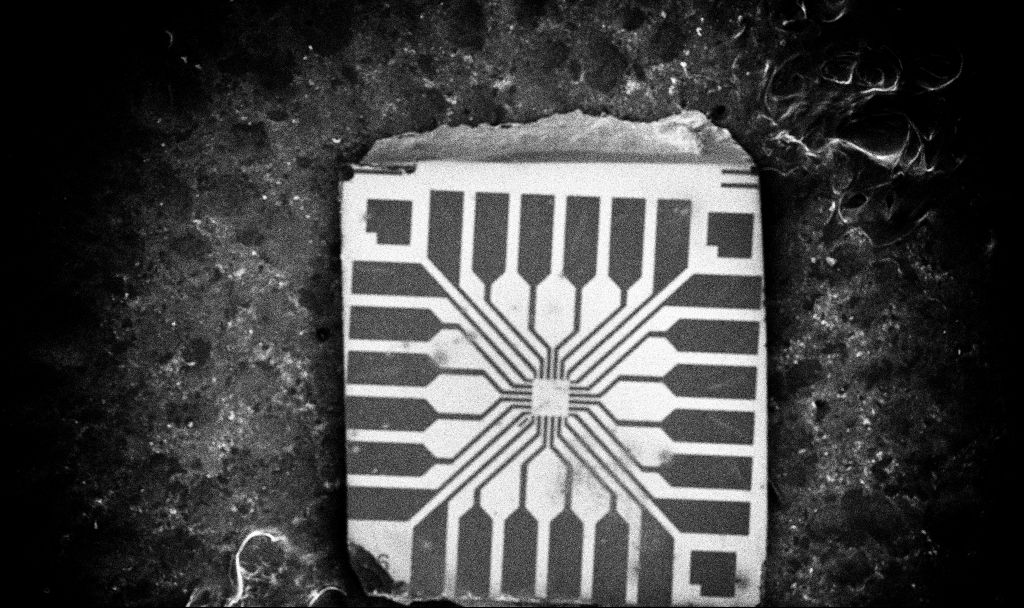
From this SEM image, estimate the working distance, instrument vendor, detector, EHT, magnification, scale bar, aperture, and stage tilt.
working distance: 10 mm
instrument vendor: Zeiss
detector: InLens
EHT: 10 kV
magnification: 0.077 K X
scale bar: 200000 nm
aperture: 30 µm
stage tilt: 0°